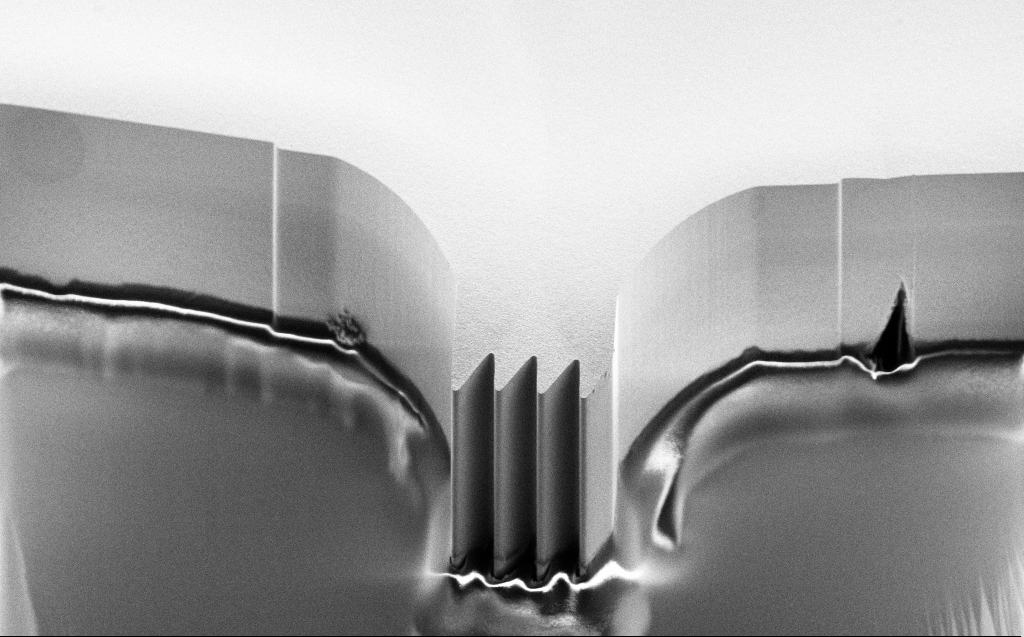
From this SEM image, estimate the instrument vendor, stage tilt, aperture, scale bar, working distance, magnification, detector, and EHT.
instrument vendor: Zeiss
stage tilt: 45°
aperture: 30 µm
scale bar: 10000 nm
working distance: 7 mm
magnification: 1.39 K X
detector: SE2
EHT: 5 kV